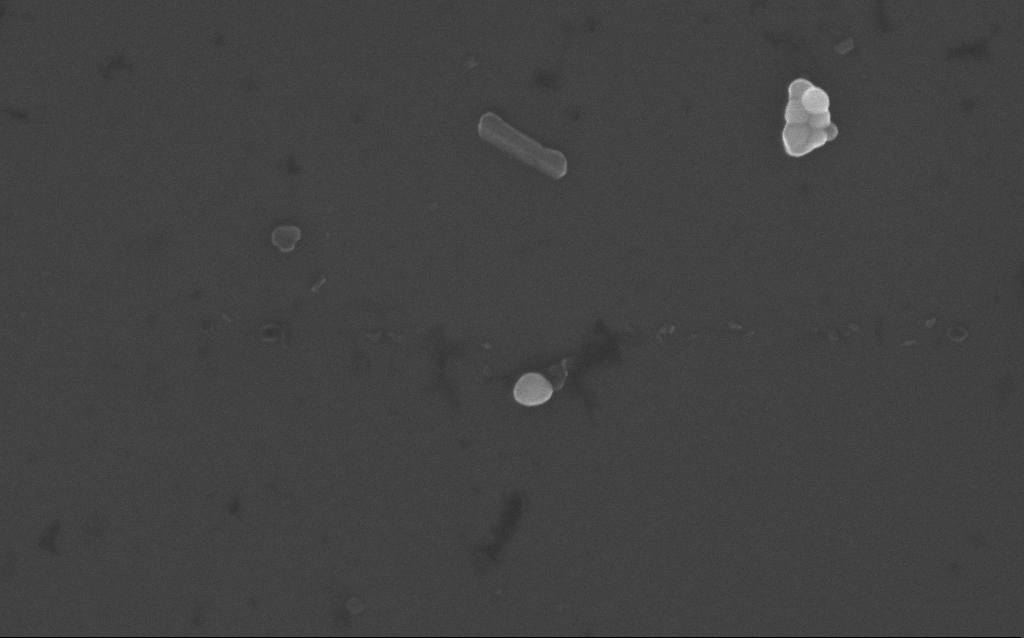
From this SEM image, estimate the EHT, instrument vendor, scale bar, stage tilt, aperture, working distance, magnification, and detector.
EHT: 10 kV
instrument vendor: Zeiss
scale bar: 1000 nm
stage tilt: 0°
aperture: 30 µm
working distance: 3 mm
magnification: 61.33 K X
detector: InLens